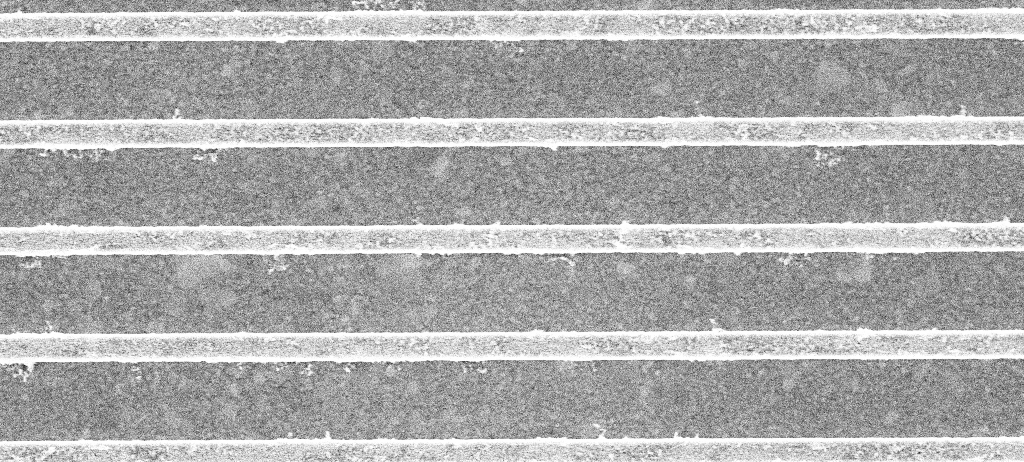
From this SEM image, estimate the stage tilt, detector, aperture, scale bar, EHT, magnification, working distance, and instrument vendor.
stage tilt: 0°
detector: InLens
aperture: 30 µm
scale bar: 1000 nm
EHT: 5 kV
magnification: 43.99 K X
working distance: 3.2 mm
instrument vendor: Zeiss